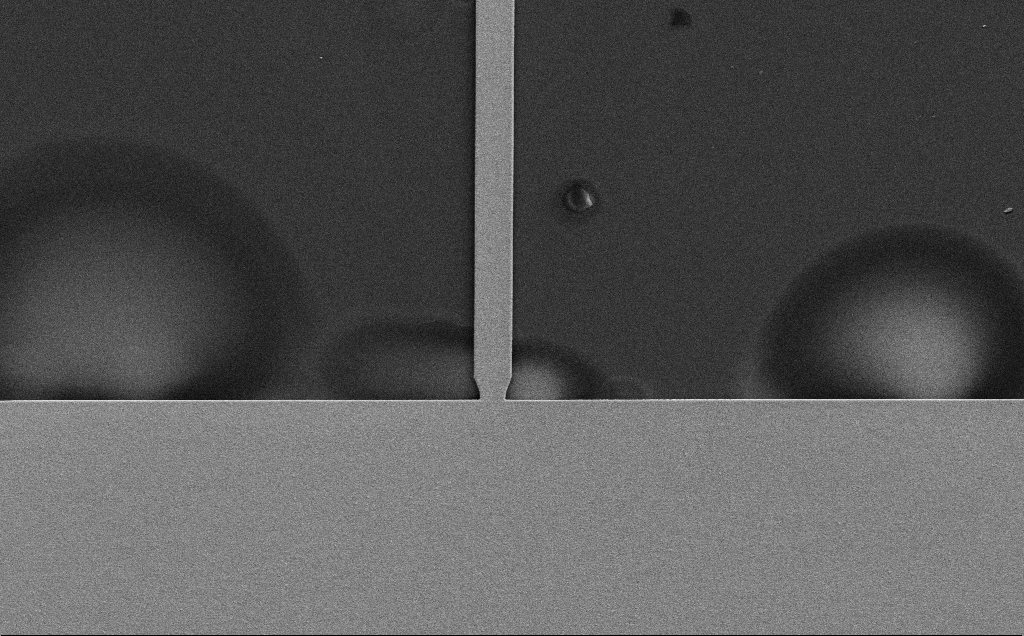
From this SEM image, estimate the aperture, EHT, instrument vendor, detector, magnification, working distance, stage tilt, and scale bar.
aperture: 30 µm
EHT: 10 kV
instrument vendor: Zeiss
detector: SE2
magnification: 1.21 K X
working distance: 12 mm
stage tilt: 0°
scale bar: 20000 nm